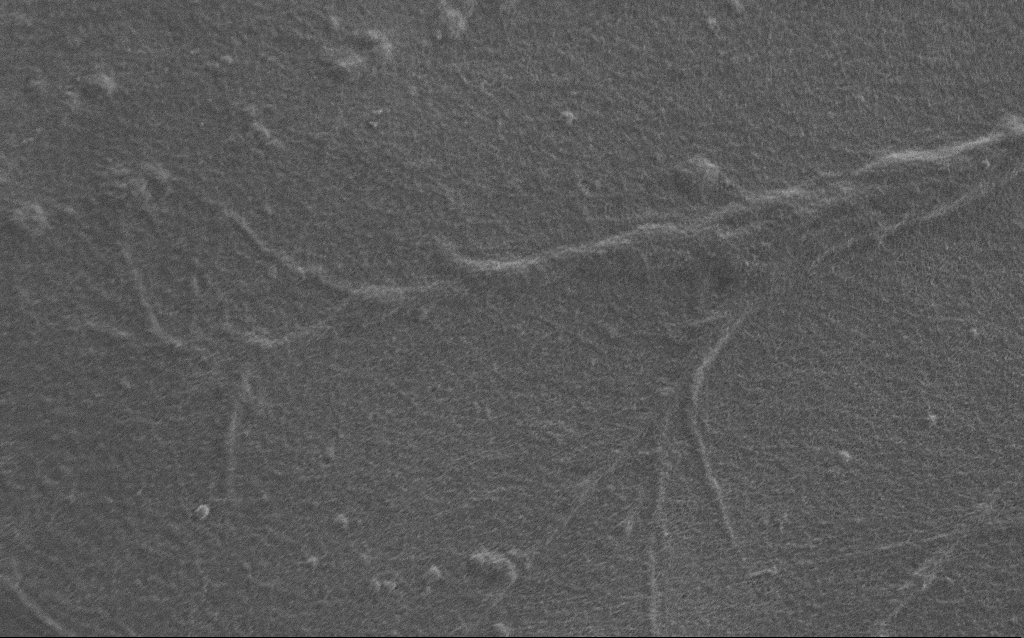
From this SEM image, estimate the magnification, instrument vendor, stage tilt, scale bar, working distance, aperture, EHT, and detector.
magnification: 7.5 K X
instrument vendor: Zeiss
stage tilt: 0°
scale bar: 2000 nm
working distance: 6 mm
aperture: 30 µm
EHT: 0.9 kV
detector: SE2